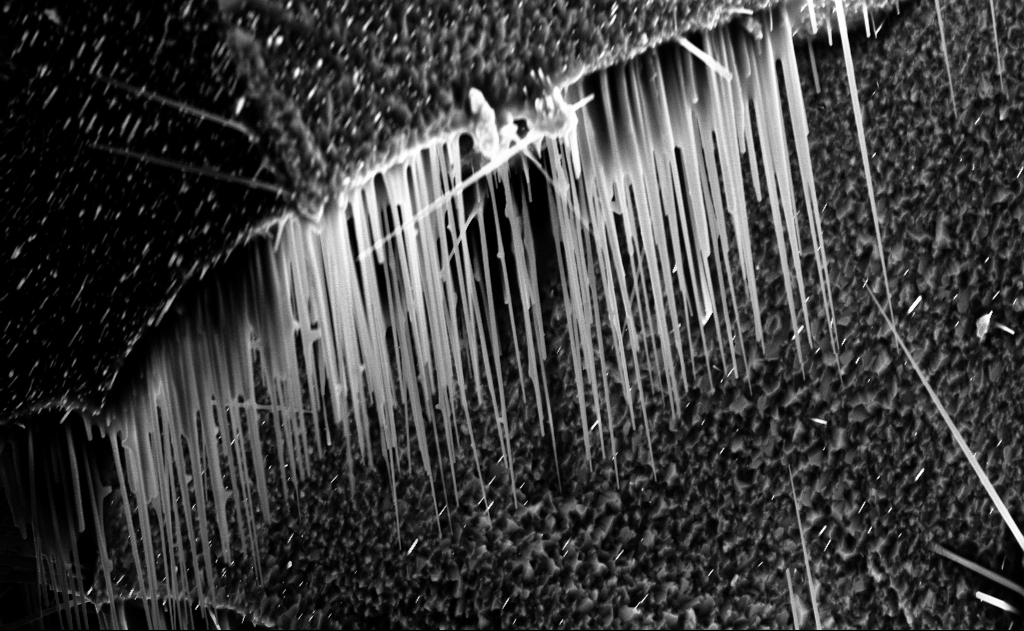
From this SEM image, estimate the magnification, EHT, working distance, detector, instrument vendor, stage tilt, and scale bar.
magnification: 9.09 K X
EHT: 10 kV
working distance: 7 mm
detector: InLens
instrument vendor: Zeiss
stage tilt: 0°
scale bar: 2000 nm